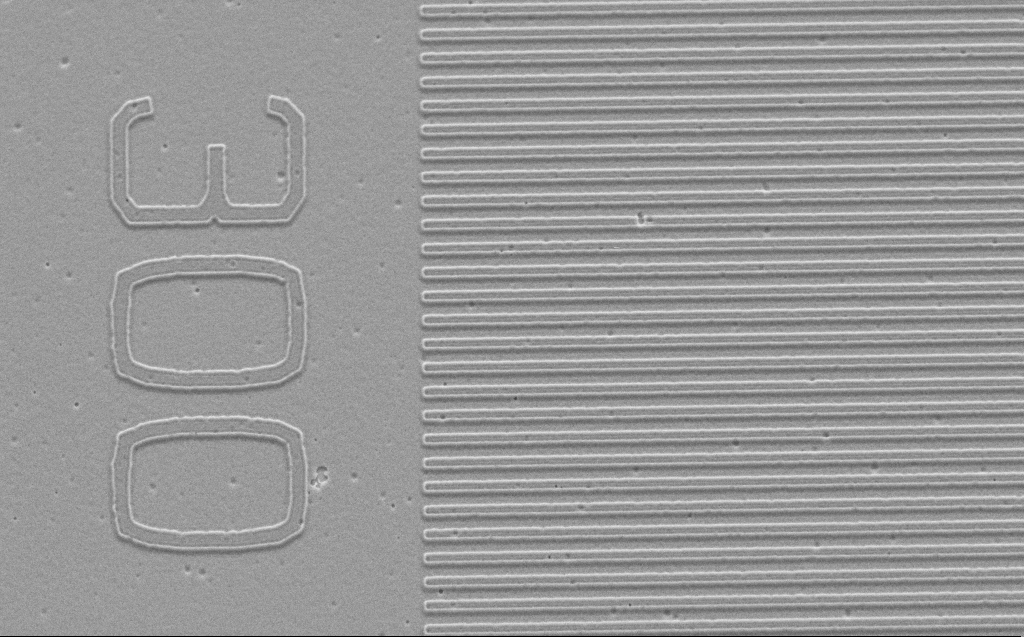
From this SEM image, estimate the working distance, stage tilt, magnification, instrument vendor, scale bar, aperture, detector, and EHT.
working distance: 9 mm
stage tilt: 41.9°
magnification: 13.95 K X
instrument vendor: Zeiss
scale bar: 2000 nm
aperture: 30 µm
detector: SE2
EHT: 3 kV